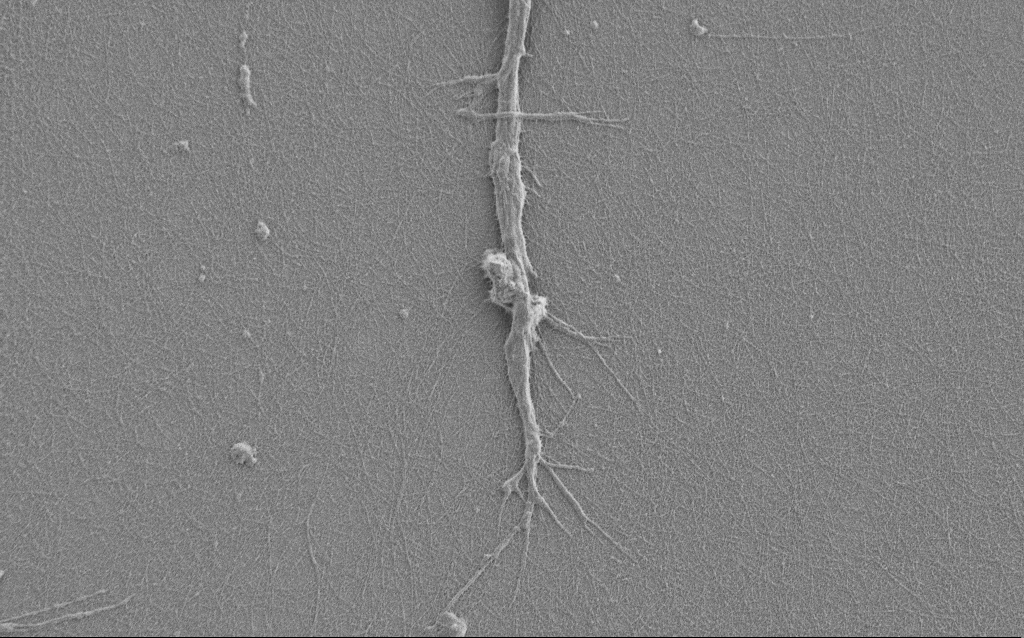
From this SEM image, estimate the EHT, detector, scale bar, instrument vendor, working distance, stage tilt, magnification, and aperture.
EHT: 1 kV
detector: SE2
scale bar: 2000 nm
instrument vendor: Zeiss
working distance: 6 mm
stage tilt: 0°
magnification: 7.5 K X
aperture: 30 µm